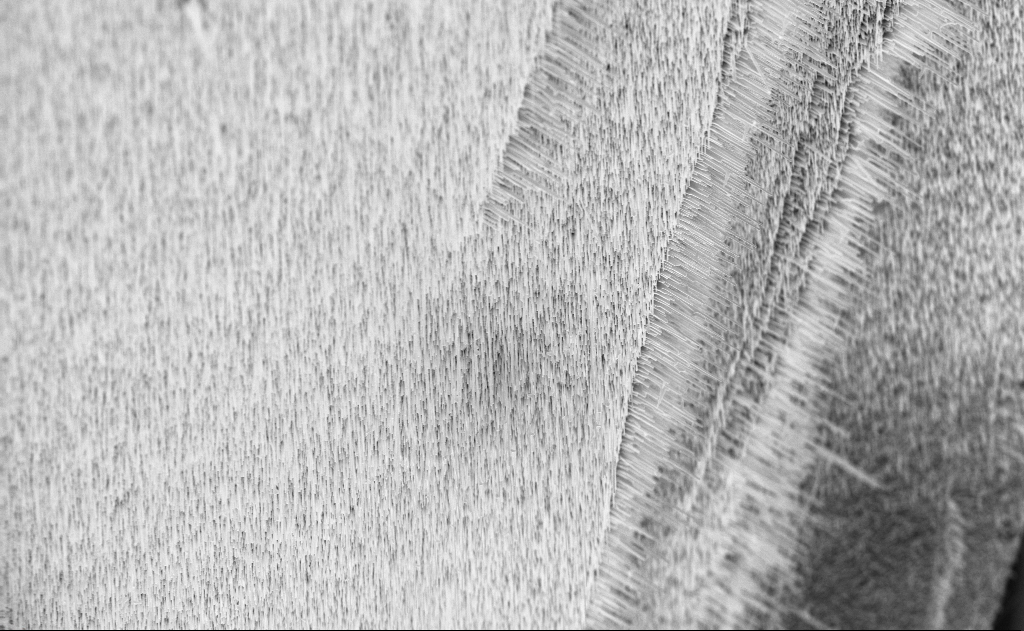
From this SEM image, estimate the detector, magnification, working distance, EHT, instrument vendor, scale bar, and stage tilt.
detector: InLens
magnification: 5 K X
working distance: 6 mm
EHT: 10 kV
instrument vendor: Zeiss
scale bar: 10000 nm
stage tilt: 0°